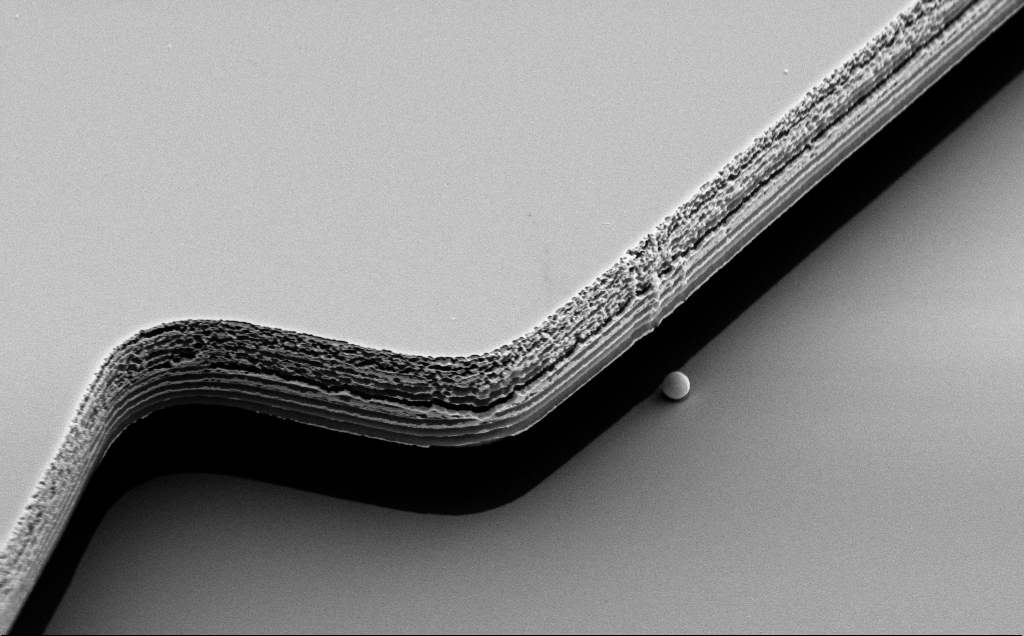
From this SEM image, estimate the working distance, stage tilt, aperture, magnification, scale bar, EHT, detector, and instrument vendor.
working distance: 10 mm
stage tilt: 50°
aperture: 30 µm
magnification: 18.14 K X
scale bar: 1000 nm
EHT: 5 kV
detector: SE2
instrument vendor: Zeiss